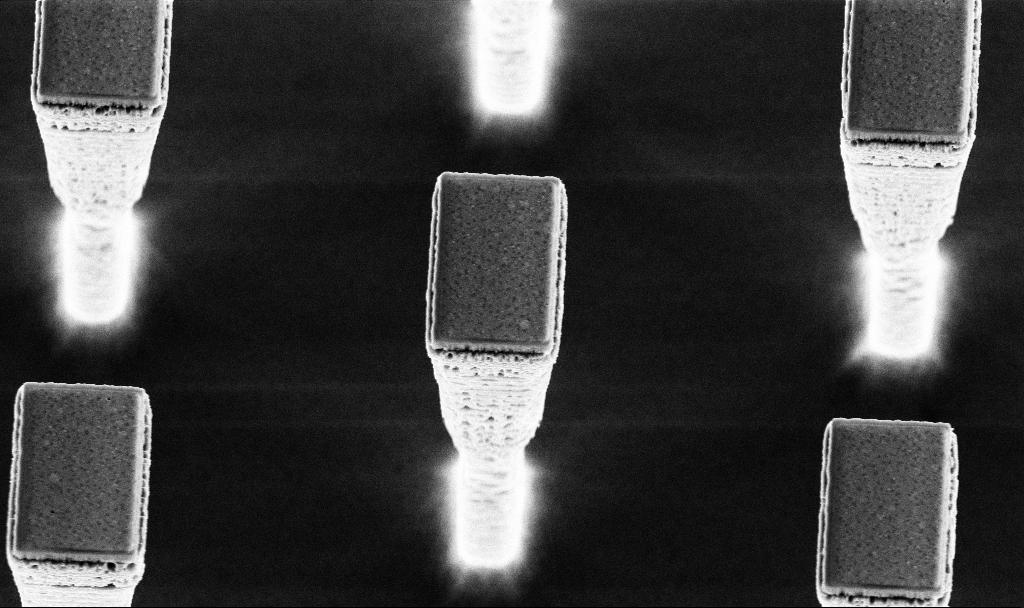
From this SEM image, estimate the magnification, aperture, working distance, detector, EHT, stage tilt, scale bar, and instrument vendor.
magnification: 14.78 K X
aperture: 30 µm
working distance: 4.1 mm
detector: InLens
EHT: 5 kV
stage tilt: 20.2°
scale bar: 2000 nm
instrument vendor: Zeiss